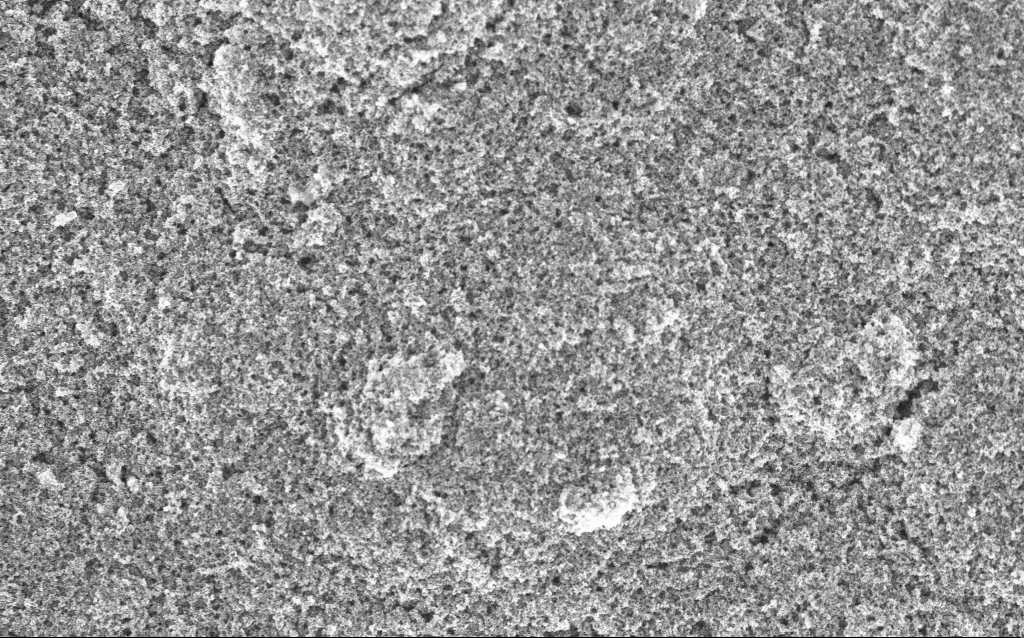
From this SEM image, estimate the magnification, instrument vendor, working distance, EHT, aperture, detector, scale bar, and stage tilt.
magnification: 20.87 K X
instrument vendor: Zeiss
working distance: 4.4 mm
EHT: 5 kV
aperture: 30 µm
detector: InLens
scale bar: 1000 nm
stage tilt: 0°